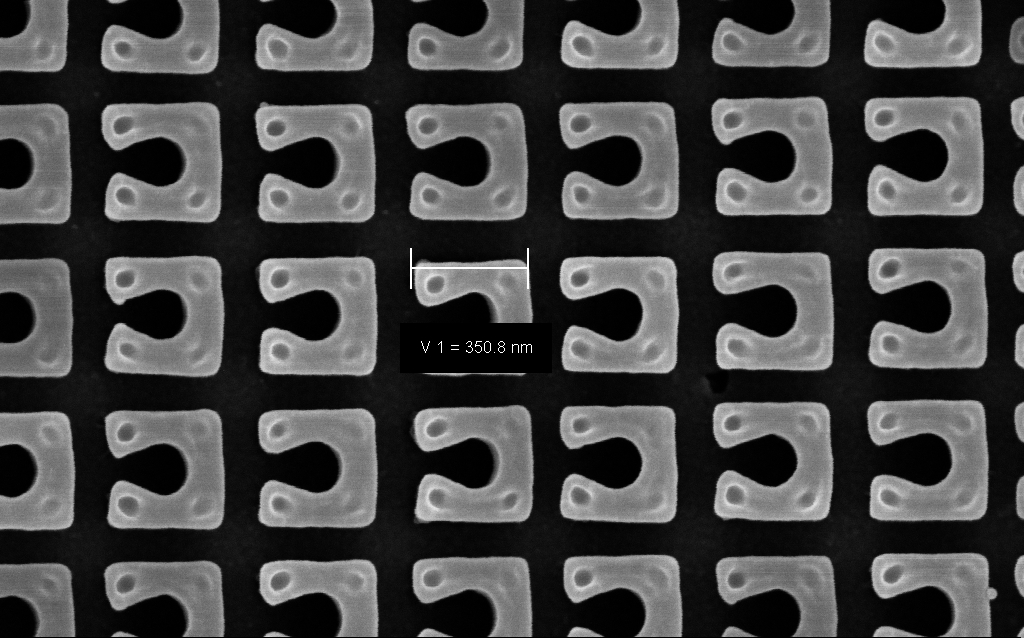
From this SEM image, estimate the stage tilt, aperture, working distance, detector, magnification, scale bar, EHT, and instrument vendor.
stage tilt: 0°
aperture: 30 µm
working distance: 4.3 mm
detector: InLens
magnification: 122.46 K X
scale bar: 200 nm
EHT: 3 kV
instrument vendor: Zeiss